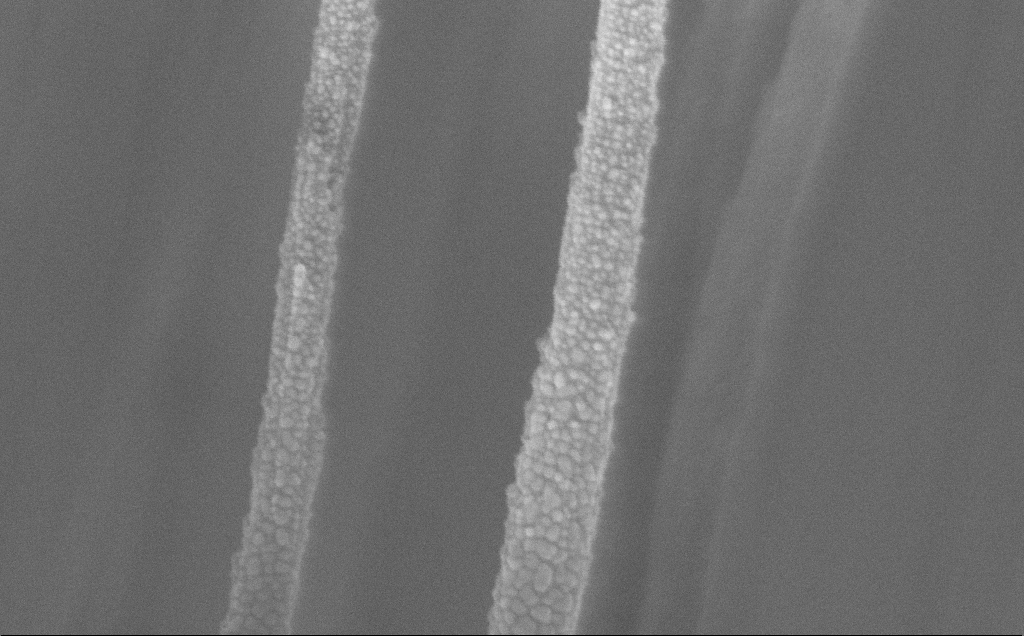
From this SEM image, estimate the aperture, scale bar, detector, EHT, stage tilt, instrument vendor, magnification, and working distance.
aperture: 30 µm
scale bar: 200 nm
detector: InLens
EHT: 5 kV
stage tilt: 0°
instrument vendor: Zeiss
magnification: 163.96 K X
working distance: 11 mm